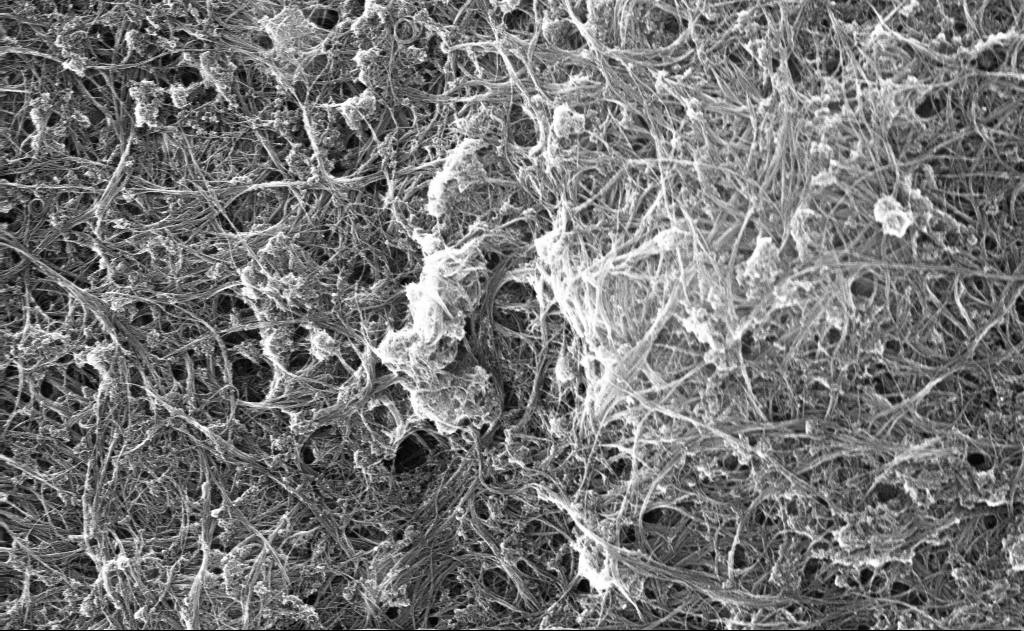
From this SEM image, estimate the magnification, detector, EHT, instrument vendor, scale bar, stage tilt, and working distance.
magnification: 40.57 K X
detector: InLens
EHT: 10 kV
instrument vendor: Zeiss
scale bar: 1000 nm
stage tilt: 0°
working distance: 6 mm